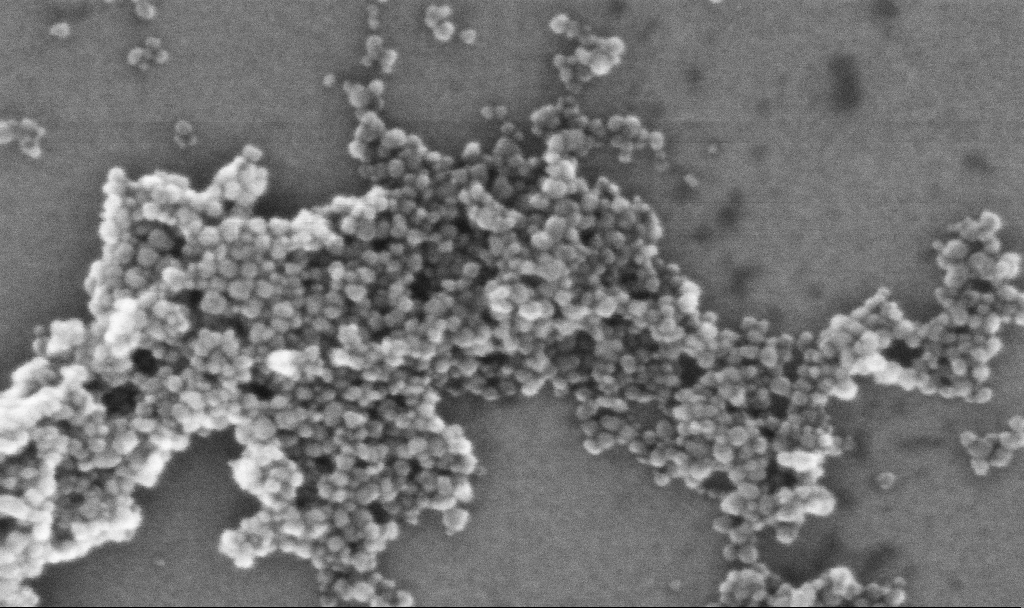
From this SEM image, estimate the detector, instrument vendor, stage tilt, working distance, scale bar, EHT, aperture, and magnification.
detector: InLens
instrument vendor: Zeiss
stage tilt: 0°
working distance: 5.4 mm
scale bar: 100 nm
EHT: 10 kV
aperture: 30 µm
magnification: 459.38 K X